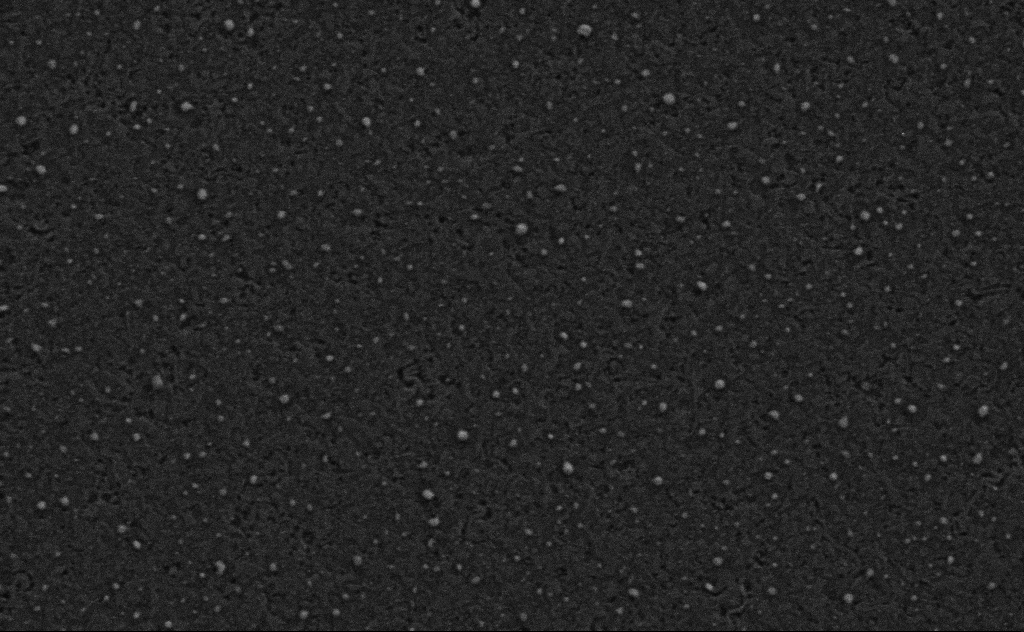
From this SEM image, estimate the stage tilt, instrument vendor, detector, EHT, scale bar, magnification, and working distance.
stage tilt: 0°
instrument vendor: Zeiss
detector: SE2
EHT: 3 kV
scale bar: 200 nm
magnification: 80 K X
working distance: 4 mm